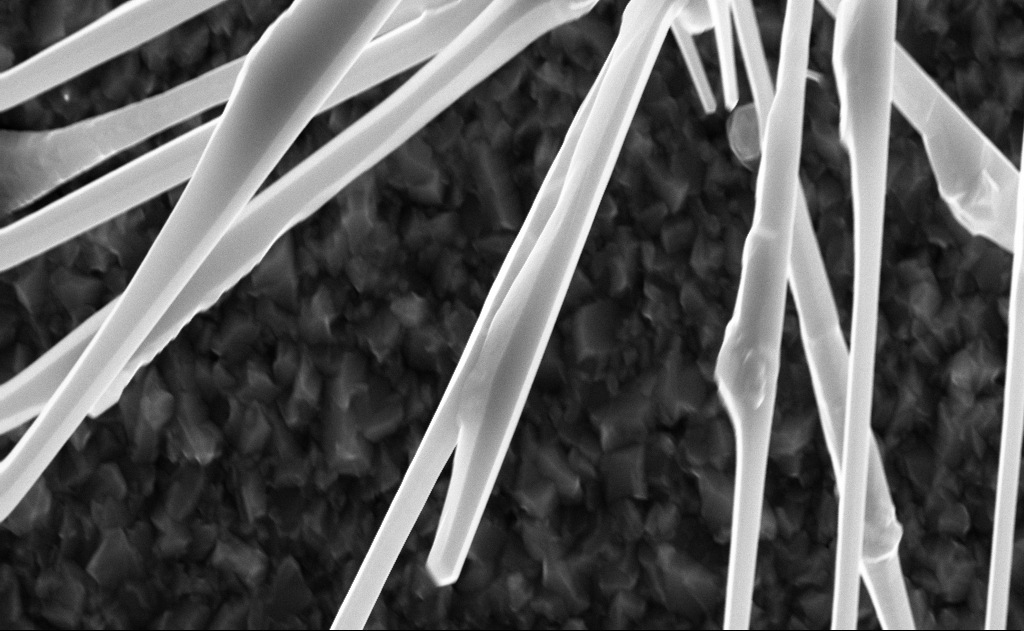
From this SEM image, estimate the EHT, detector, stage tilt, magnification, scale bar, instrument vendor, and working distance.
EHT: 10 kV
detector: InLens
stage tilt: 0°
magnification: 40 K X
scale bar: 1000 nm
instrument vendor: Zeiss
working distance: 6 mm